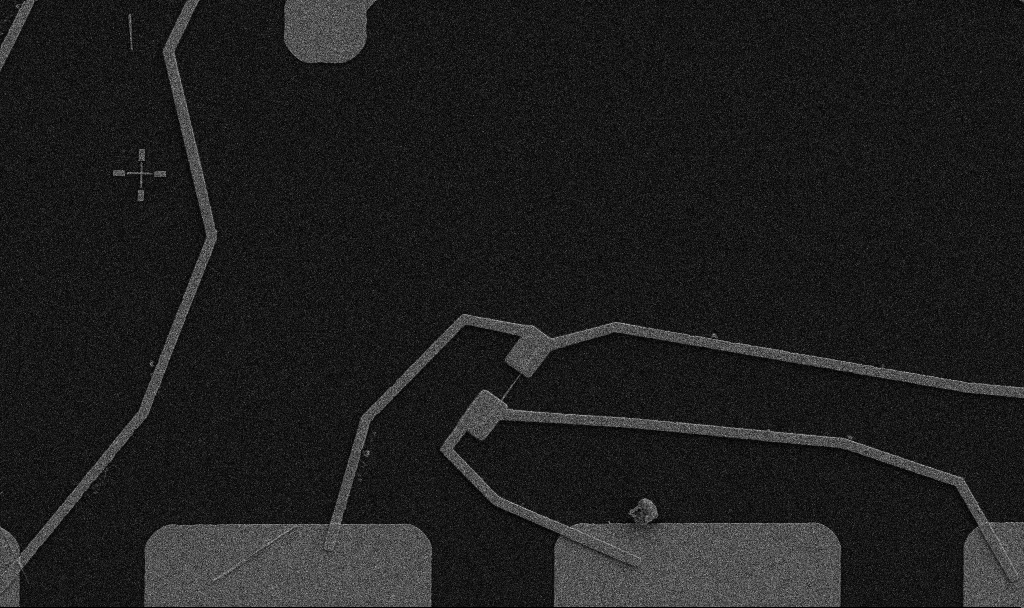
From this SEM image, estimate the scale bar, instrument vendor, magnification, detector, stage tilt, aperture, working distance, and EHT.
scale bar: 10000 nm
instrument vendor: Zeiss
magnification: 5 K X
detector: SE2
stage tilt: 0°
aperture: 30 µm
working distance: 10.7 mm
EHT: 5 kV